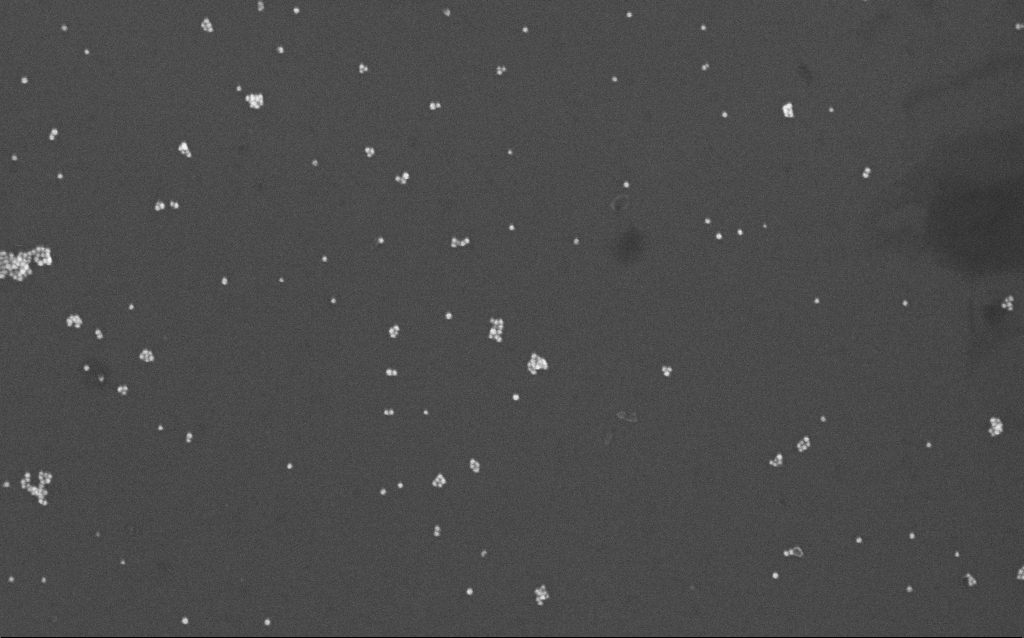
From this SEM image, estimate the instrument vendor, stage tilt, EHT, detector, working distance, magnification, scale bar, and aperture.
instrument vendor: Zeiss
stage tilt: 0°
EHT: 10 kV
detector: InLens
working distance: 7 mm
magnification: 100 K X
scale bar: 200 nm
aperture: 30 µm